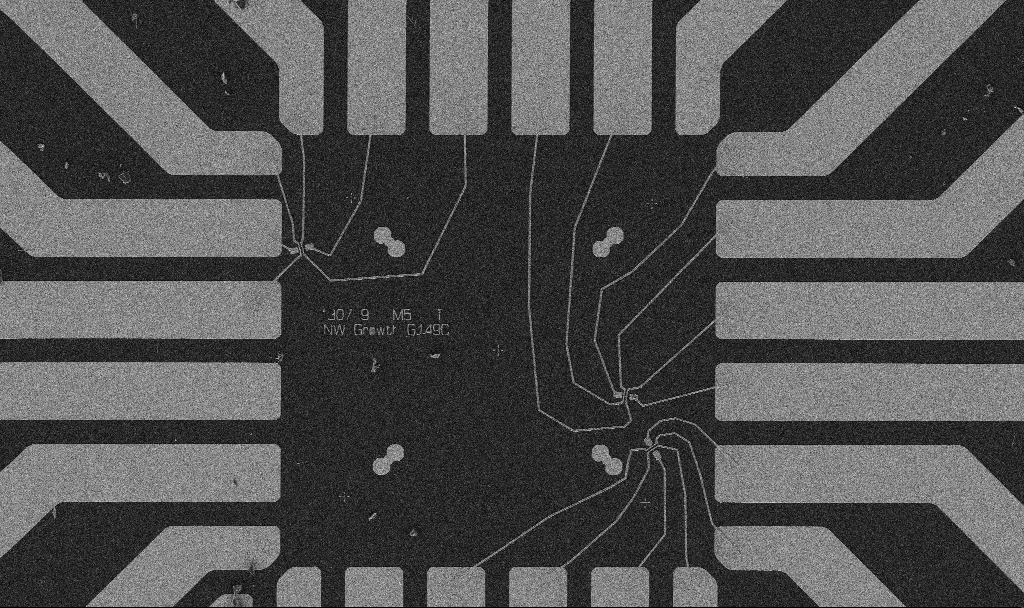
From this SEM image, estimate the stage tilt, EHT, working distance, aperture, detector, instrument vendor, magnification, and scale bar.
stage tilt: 0°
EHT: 5 kV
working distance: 10.7 mm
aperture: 30 µm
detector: SE2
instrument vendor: Zeiss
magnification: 1 K X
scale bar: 20000 nm